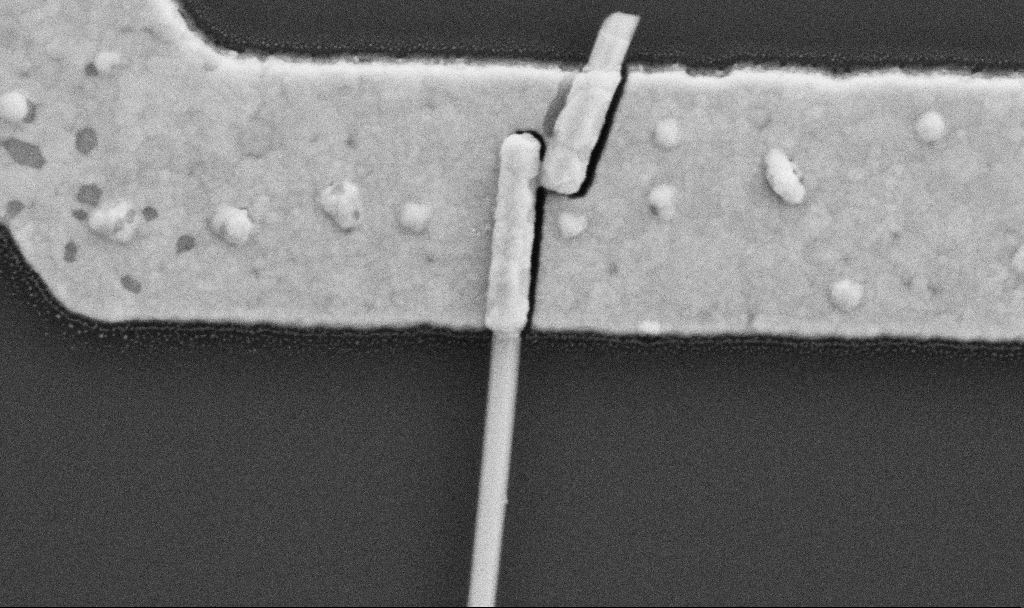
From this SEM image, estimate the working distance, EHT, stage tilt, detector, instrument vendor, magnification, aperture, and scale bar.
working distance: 8.7 mm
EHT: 5 kV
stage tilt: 0°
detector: SE2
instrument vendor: Zeiss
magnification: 100 K X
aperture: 30 µm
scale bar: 200 nm